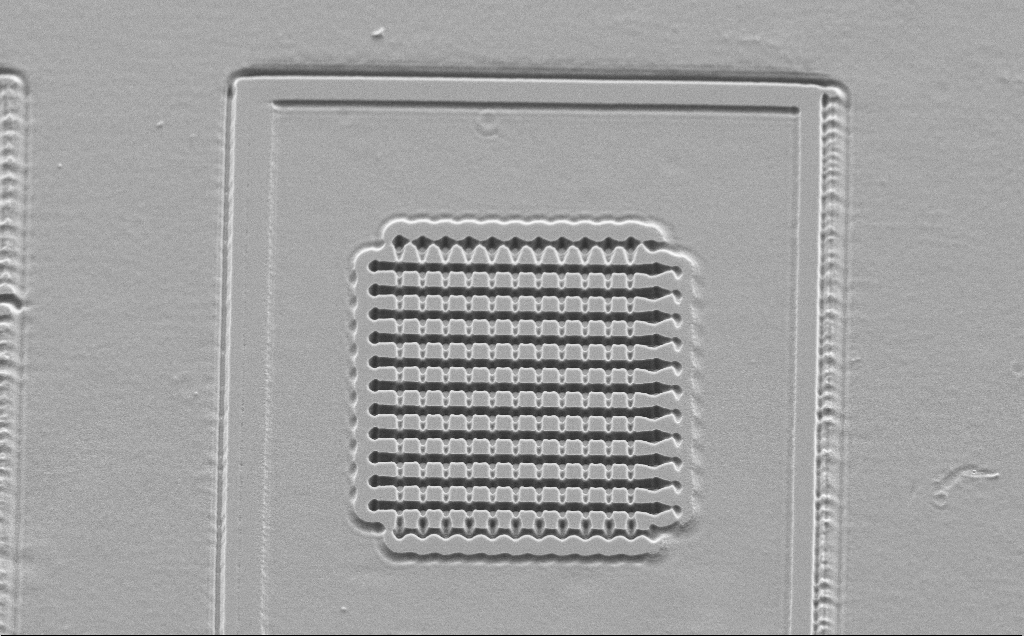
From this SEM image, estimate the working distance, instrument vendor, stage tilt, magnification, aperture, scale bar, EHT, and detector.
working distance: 10 mm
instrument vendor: Zeiss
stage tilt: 45°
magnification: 2.13 K X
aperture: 30 µm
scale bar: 20000 nm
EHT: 5 kV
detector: SE2